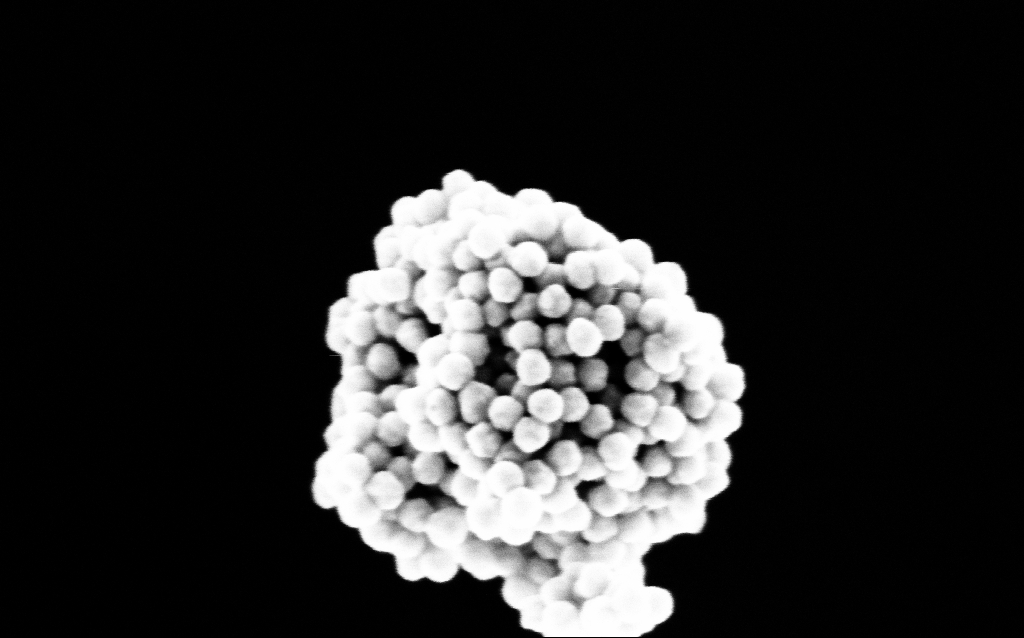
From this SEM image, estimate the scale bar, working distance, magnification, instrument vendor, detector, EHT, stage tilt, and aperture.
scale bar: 100 nm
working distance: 3.3 mm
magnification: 216.02 K X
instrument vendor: Zeiss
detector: InLens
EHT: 5 kV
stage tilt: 0°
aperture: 30 µm